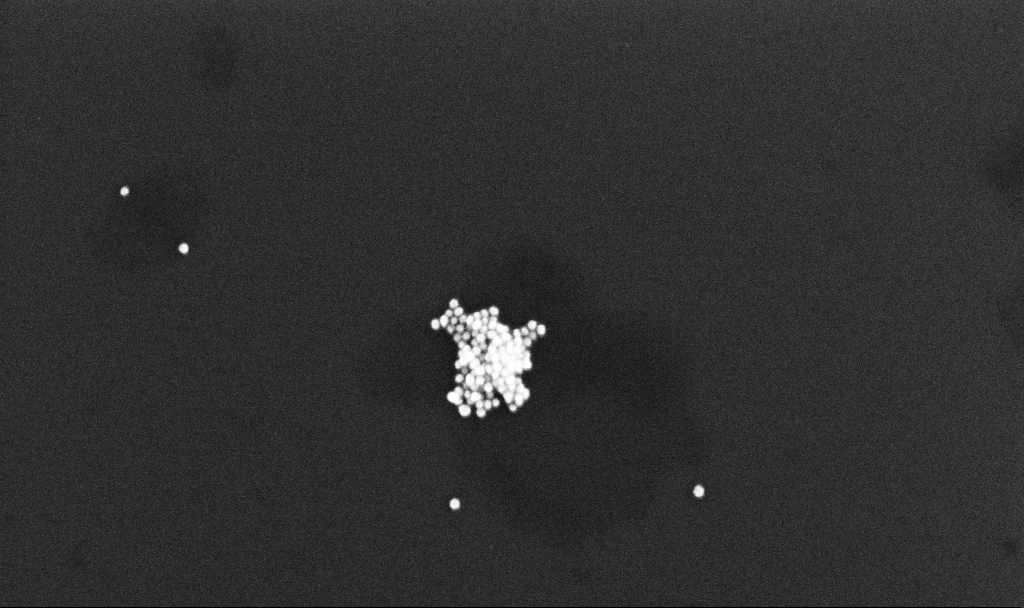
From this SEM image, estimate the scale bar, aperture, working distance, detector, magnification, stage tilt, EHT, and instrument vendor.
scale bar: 200 nm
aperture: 30 µm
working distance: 3.2 mm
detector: InLens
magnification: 157.95 K X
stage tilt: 0°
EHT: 10 kV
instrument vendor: Zeiss